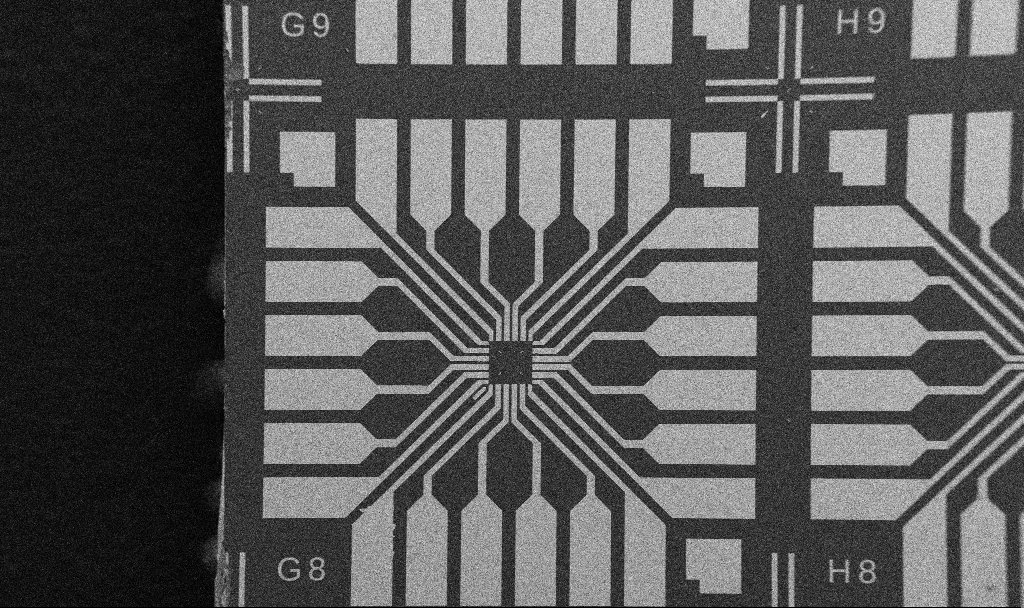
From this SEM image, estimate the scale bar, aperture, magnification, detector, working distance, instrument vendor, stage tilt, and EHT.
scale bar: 200000 nm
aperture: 30 µm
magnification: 0.1 K X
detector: SE2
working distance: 10.7 mm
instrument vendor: Zeiss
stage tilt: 0°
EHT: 5 kV